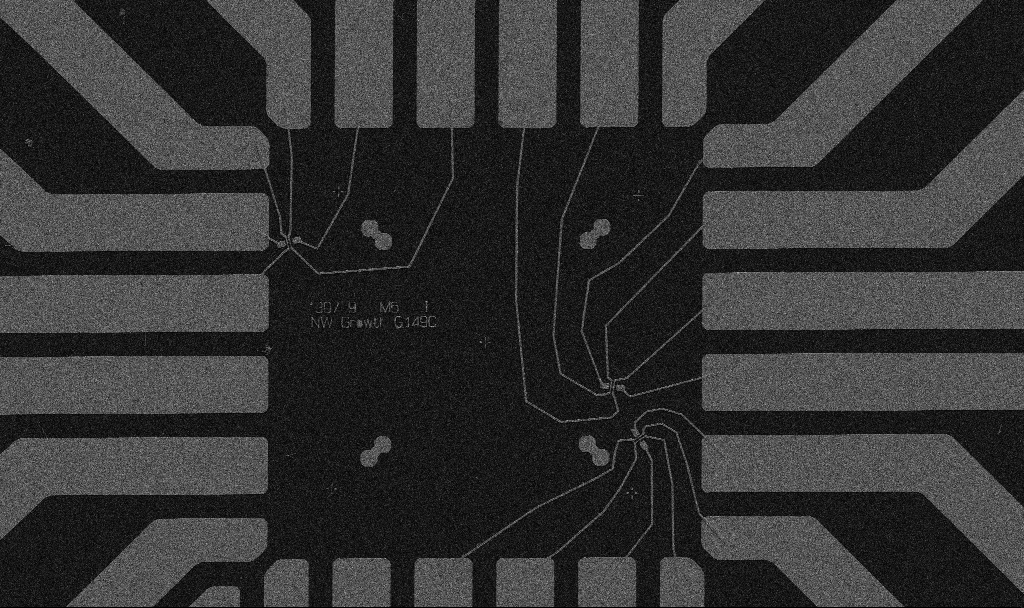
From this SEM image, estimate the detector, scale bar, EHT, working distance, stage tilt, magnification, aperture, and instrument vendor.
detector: SE2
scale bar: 20000 nm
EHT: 5 kV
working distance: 10.7 mm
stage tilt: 0°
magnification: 1 K X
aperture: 30 µm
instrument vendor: Zeiss